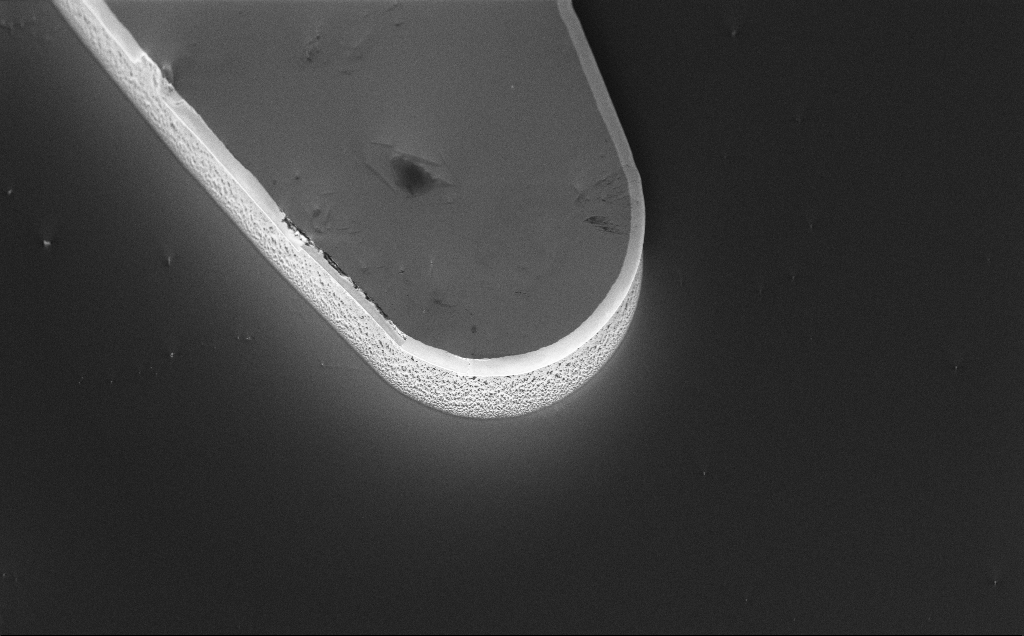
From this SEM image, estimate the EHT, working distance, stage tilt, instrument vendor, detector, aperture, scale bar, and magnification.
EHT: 5 kV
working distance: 8 mm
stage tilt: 45°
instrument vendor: Zeiss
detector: InLens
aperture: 30 µm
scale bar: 10000 nm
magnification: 1.43 K X